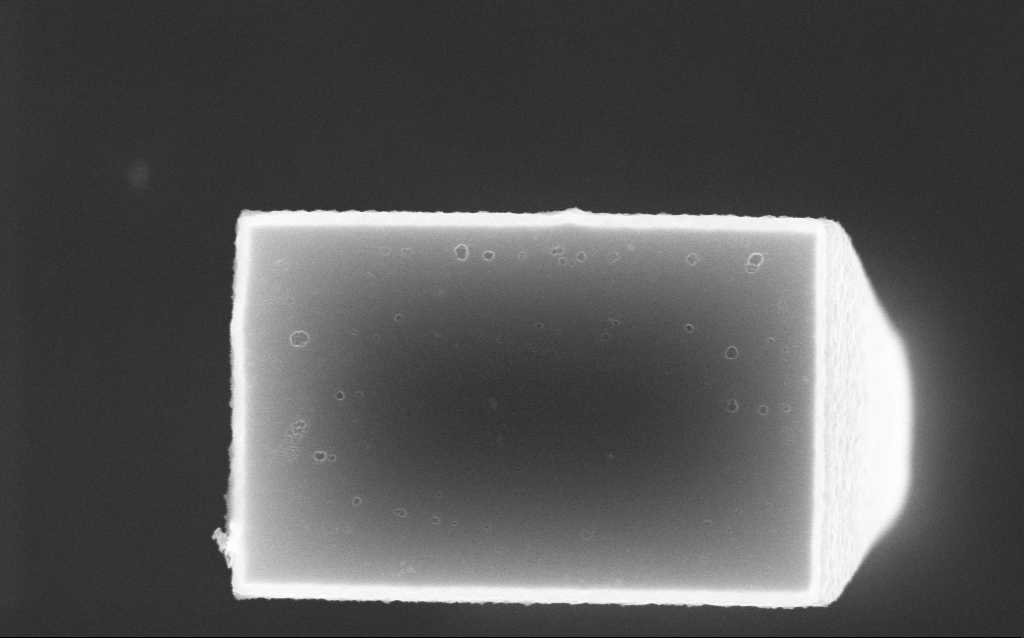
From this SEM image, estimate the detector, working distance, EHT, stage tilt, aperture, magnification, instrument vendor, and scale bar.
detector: InLens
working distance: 8.3 mm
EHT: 10 kV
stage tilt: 0°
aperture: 30 µm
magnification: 77.66 K X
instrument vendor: Zeiss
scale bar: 200 nm